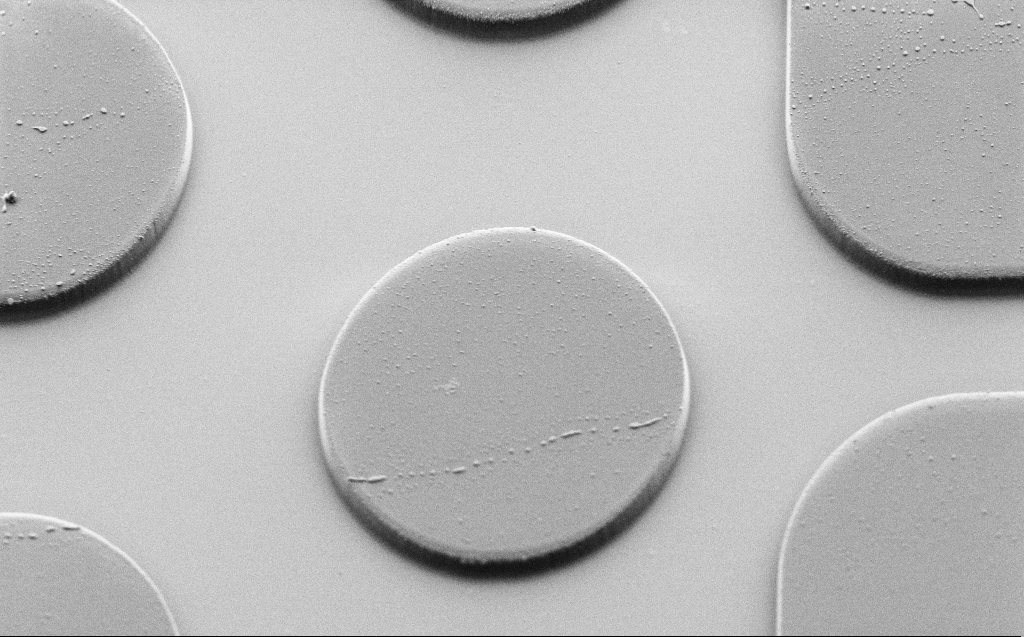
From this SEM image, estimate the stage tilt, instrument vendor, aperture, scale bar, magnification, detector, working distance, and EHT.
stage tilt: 45°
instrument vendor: Zeiss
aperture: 30 µm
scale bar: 20000 nm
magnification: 3.43 K X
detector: SE2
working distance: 6 mm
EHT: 1.7 kV